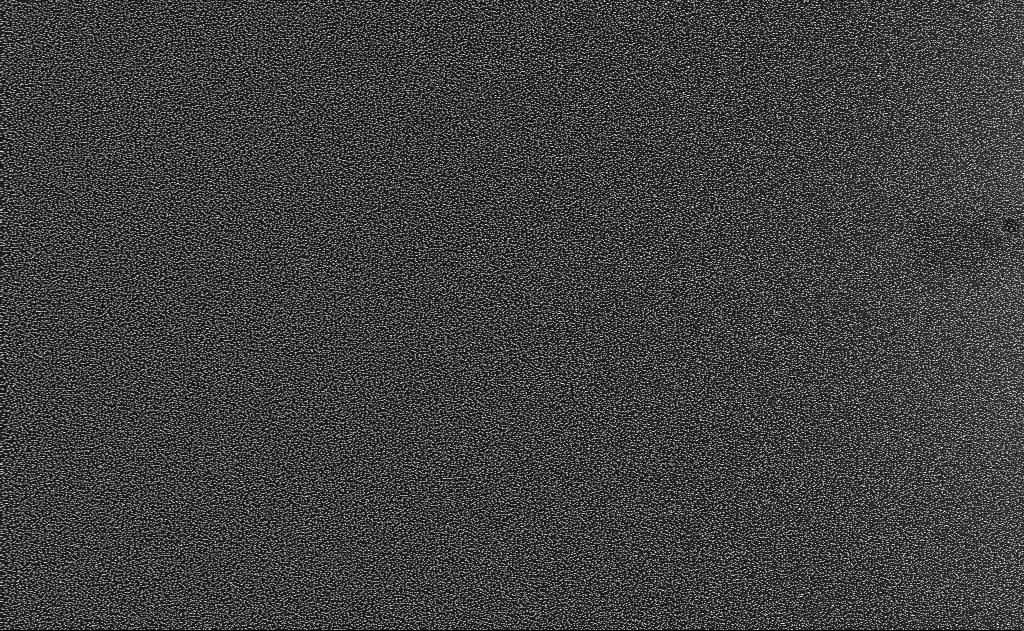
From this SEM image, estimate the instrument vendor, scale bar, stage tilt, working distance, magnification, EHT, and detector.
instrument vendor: Zeiss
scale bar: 10000 nm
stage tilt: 0°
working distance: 11 mm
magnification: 5 K X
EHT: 10 kV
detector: InLens